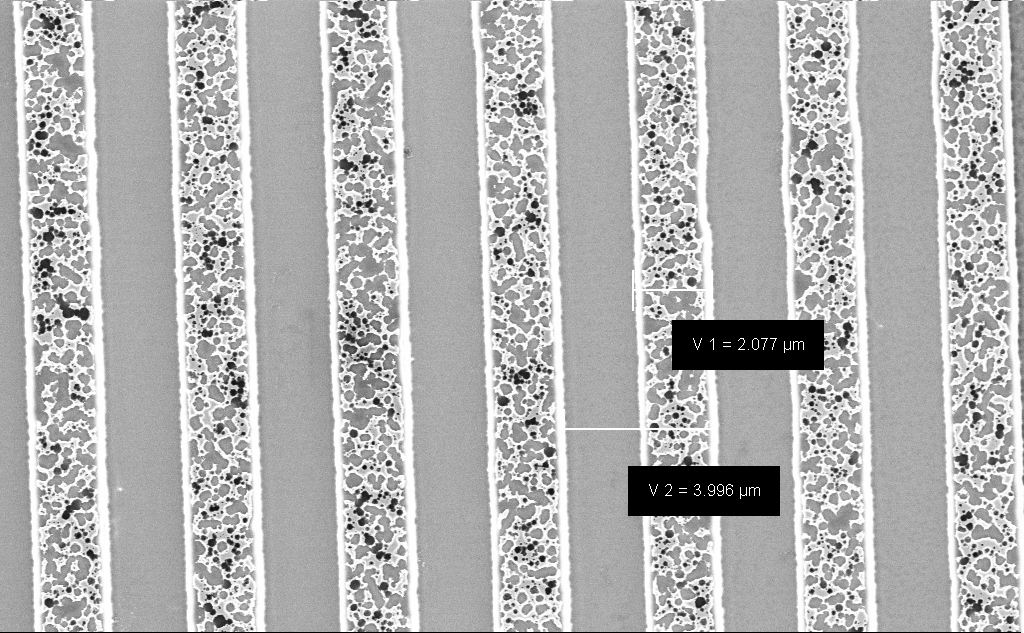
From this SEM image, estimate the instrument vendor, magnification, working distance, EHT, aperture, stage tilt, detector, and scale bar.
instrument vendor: Zeiss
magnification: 13.97 K X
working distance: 7.9 mm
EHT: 3 kV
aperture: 30 µm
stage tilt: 0°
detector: InLens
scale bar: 2000 nm